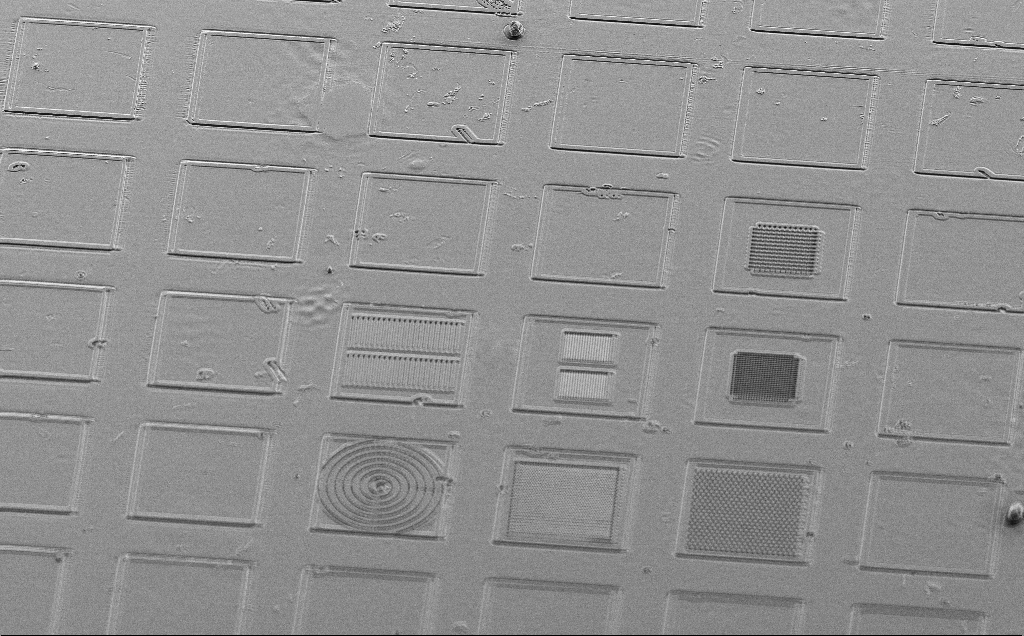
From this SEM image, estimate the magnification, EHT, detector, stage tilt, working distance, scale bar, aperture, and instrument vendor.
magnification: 0.474 K X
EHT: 5 kV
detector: SE2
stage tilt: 45°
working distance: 10 mm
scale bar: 100000 nm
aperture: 30 µm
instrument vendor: Zeiss